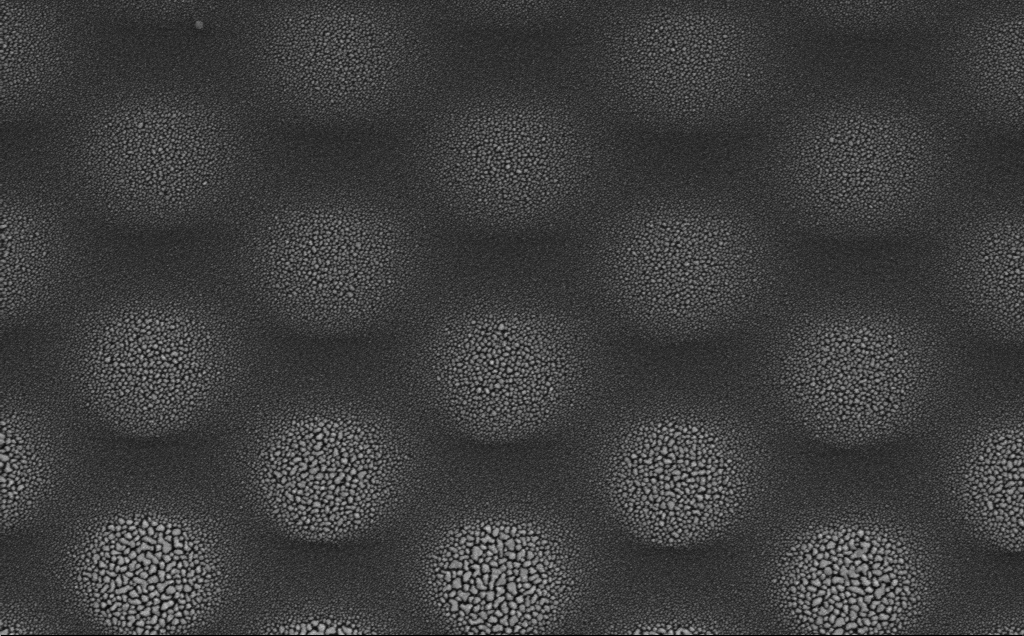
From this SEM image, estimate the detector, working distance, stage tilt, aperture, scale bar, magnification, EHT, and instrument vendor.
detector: SE2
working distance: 20 mm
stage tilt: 0°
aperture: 30 µm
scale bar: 1000 nm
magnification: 24.49 K X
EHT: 5 kV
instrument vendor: Zeiss